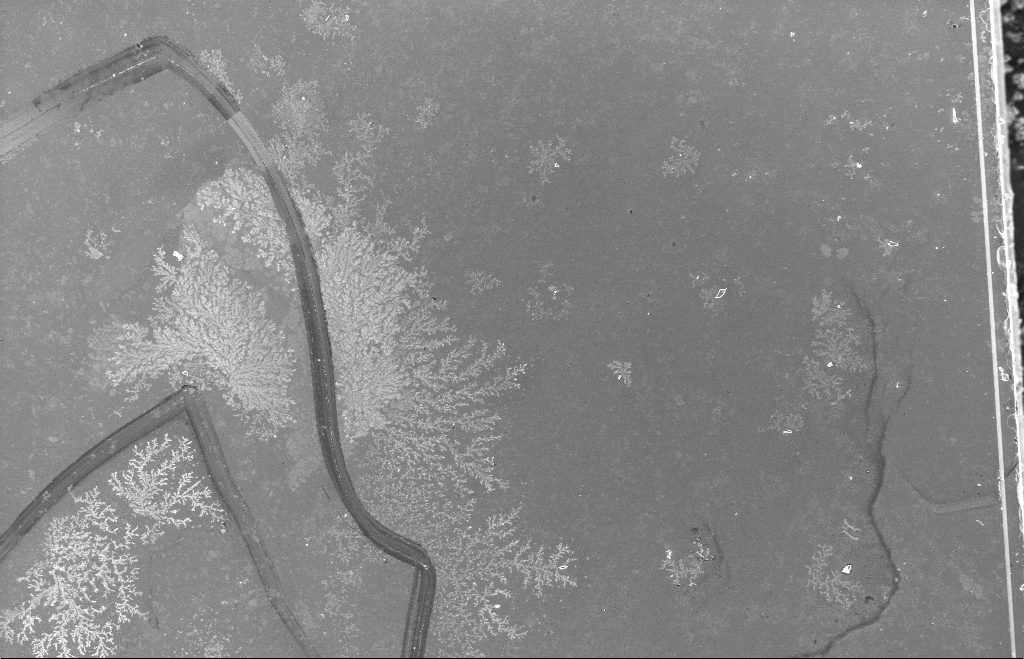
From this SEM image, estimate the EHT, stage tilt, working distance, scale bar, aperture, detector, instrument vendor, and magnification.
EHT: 10 kV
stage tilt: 0°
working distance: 8 mm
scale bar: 100000 nm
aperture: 30 µm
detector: InLens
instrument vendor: Zeiss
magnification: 0.351 K X